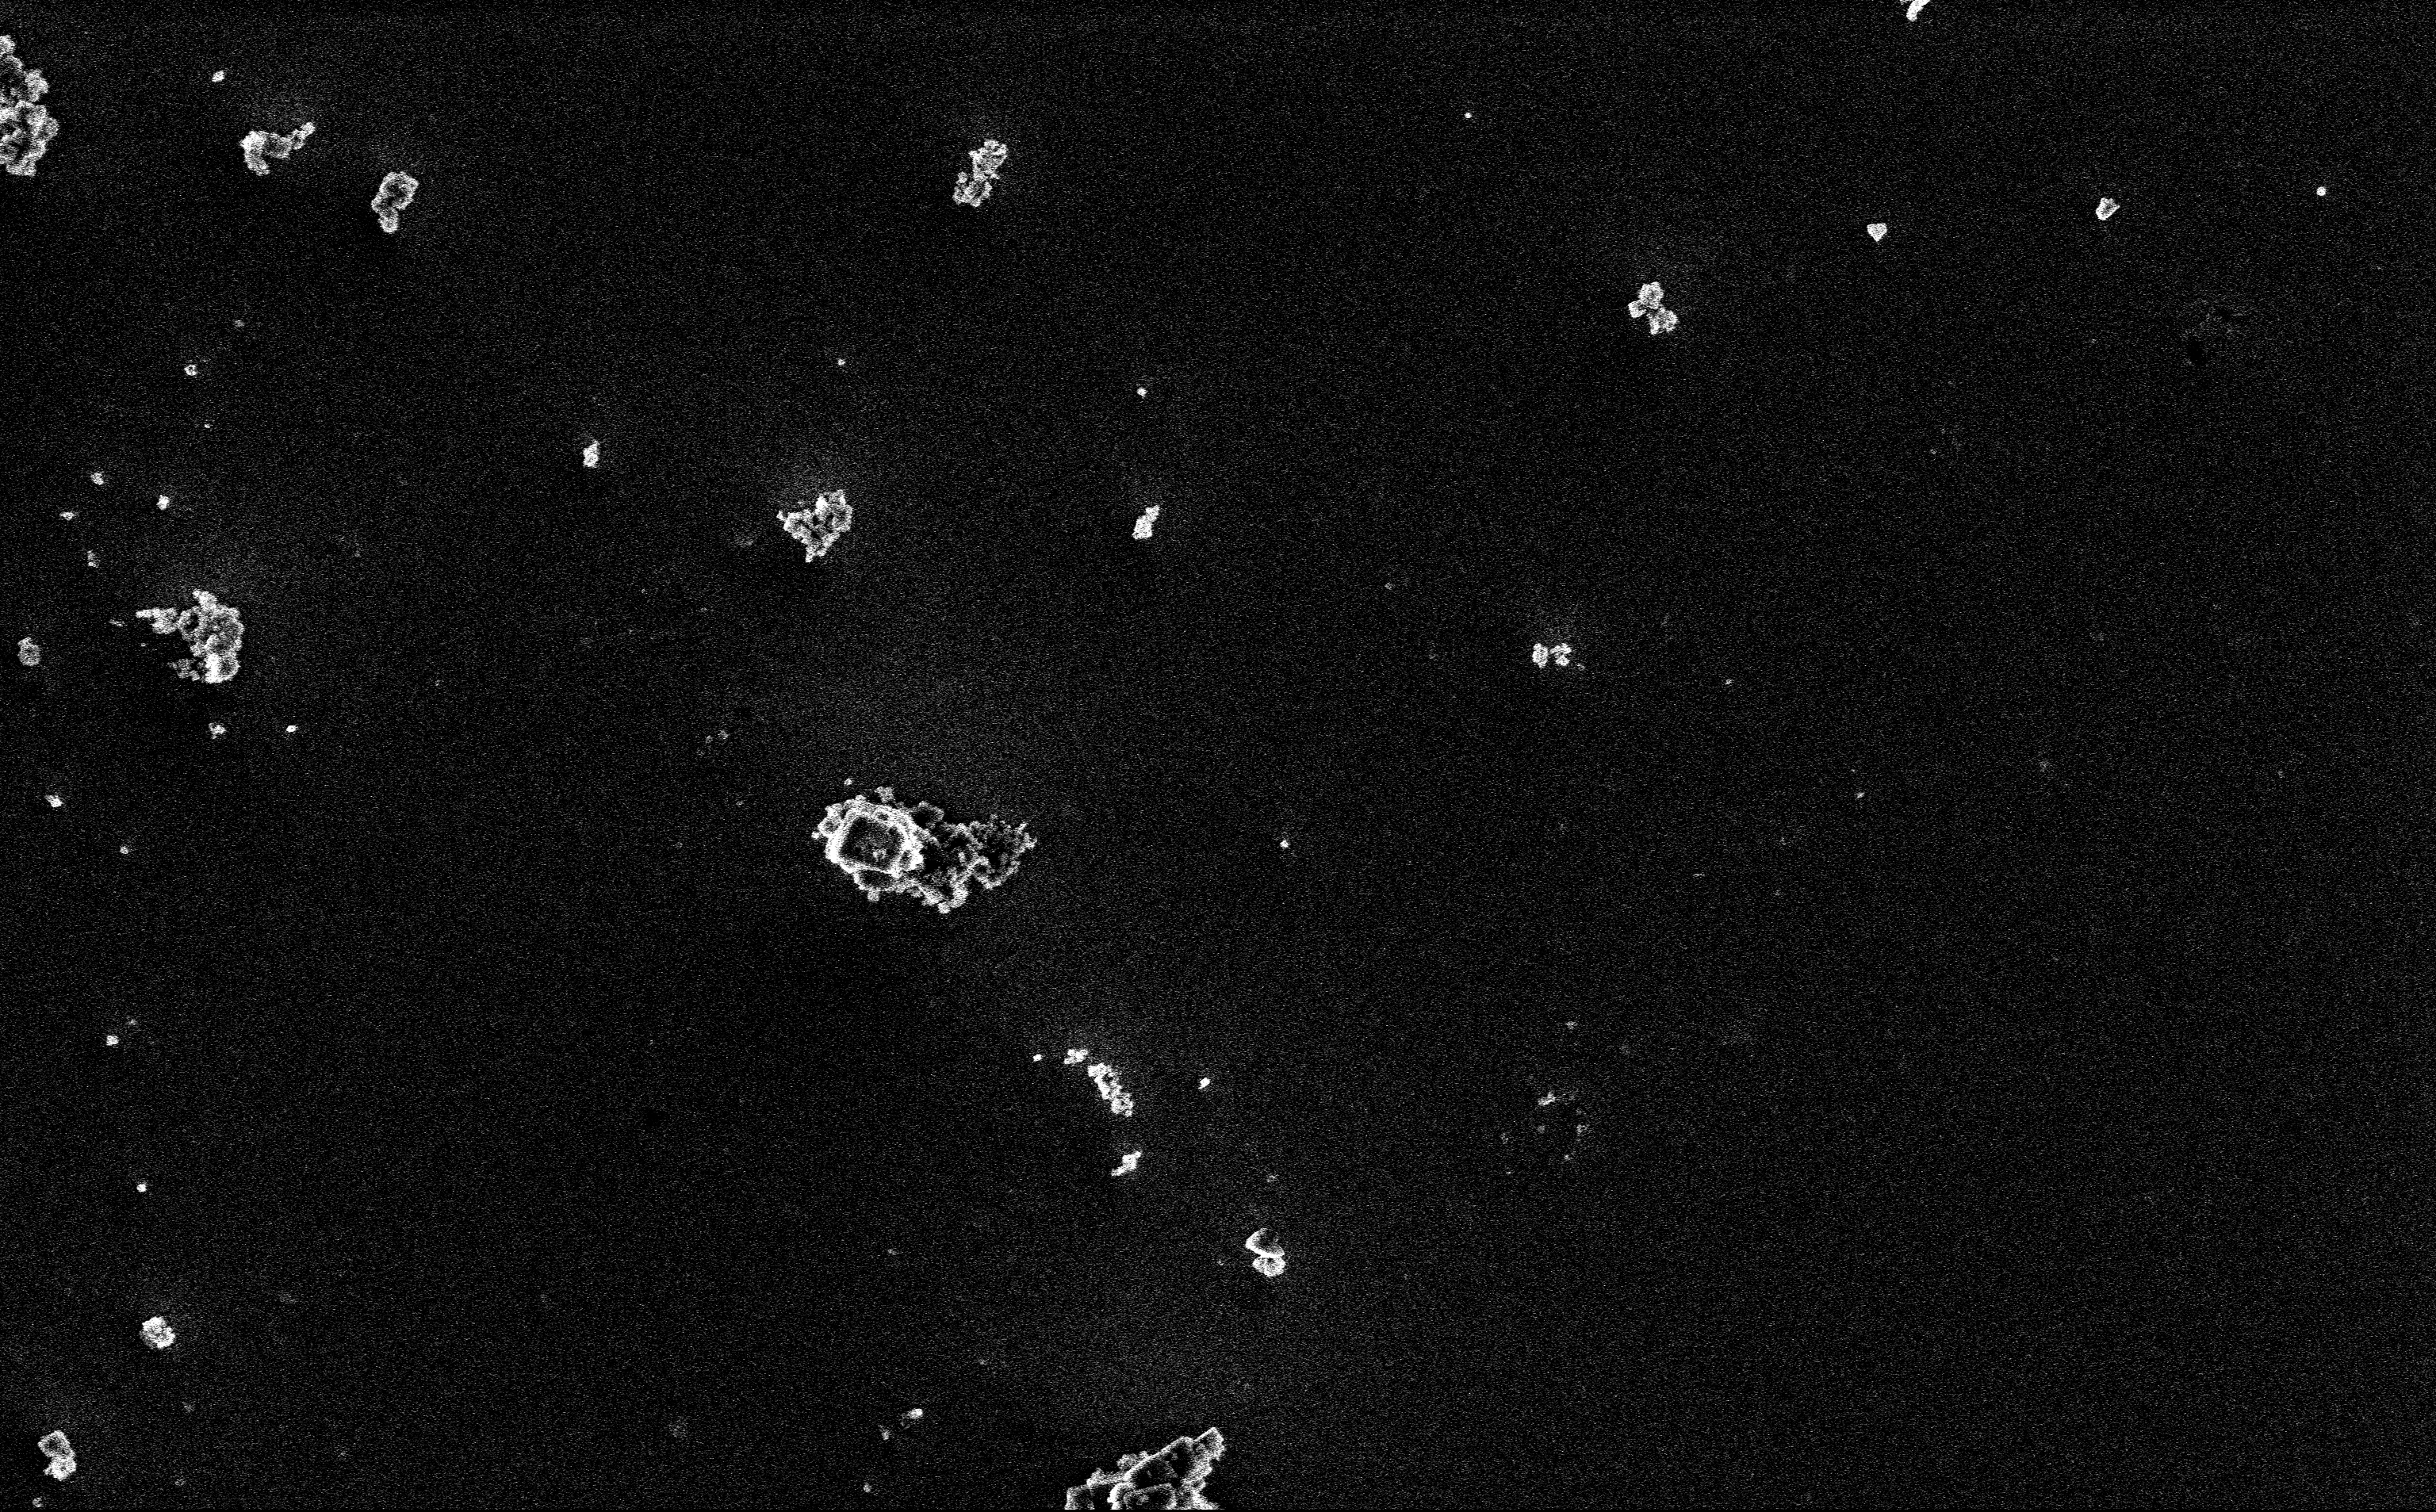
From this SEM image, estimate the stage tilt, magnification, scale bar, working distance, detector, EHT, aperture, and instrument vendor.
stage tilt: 0°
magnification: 12.85 K X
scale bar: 2000 nm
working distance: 3 mm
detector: InLens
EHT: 3 kV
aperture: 30 µm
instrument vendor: Zeiss